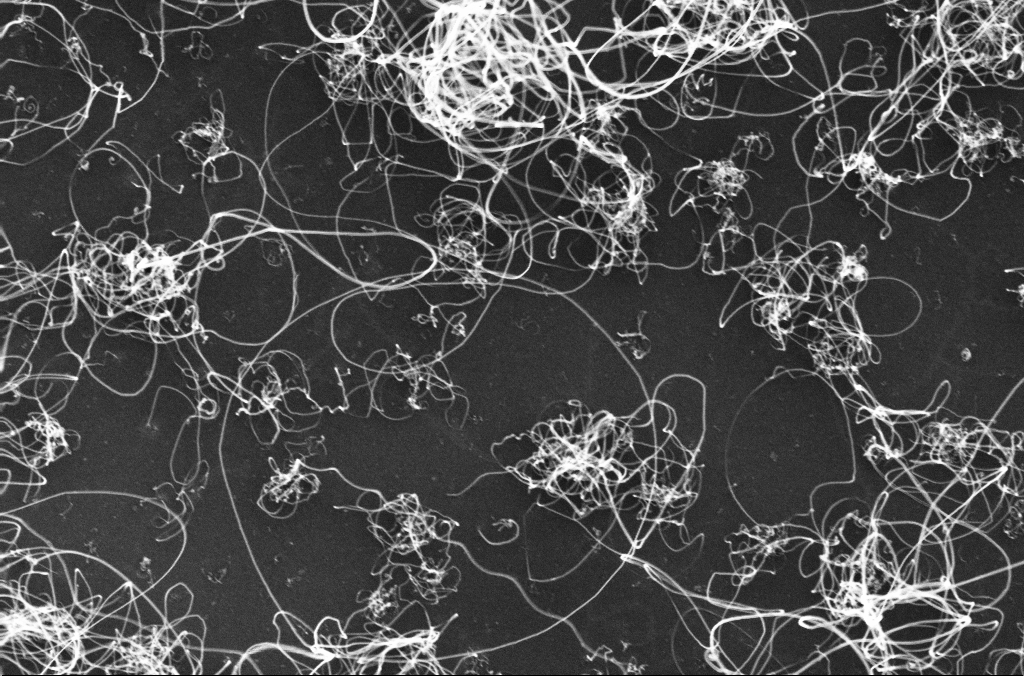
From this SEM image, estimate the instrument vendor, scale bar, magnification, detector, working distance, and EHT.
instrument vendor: Zeiss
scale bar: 1000 nm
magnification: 50 K X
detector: InLens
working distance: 3.3 mm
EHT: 10 kV